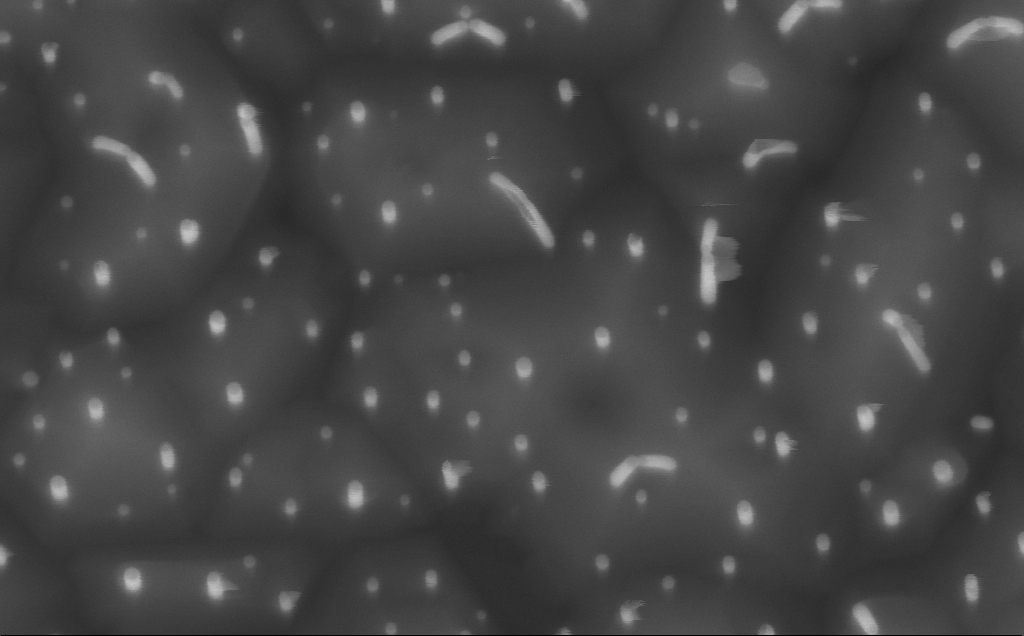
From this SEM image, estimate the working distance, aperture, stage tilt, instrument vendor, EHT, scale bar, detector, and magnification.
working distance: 5 mm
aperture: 30 µm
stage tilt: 0°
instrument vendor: Zeiss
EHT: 10 kV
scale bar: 100 nm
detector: InLens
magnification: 150 K X